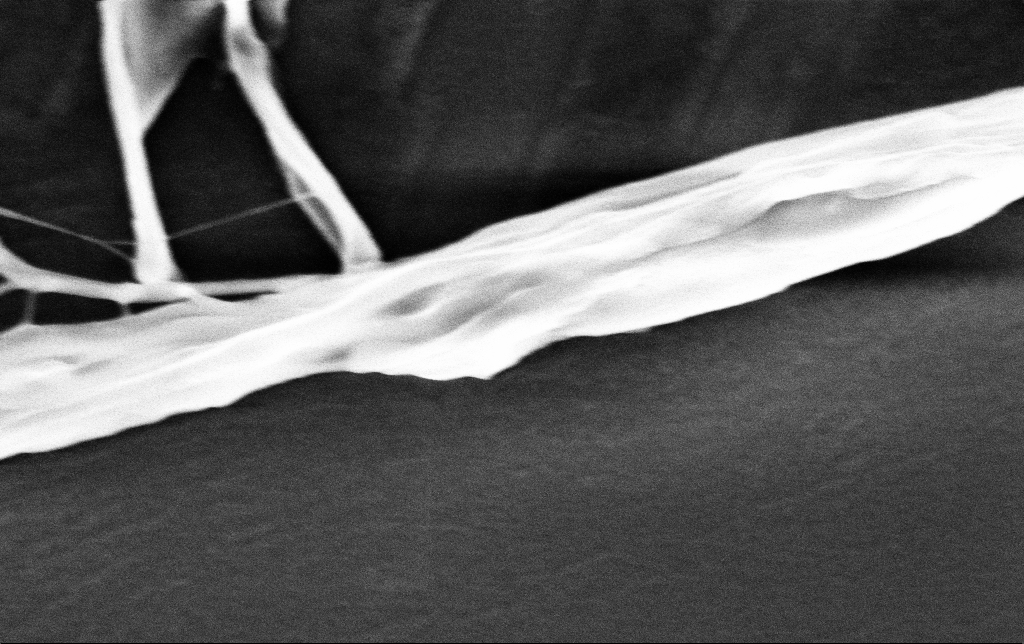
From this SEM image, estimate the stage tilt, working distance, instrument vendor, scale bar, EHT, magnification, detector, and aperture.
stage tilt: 0°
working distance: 3.5 mm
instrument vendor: Zeiss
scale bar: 200 nm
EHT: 3 kV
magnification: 74.86 K X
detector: InLens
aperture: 30 µm